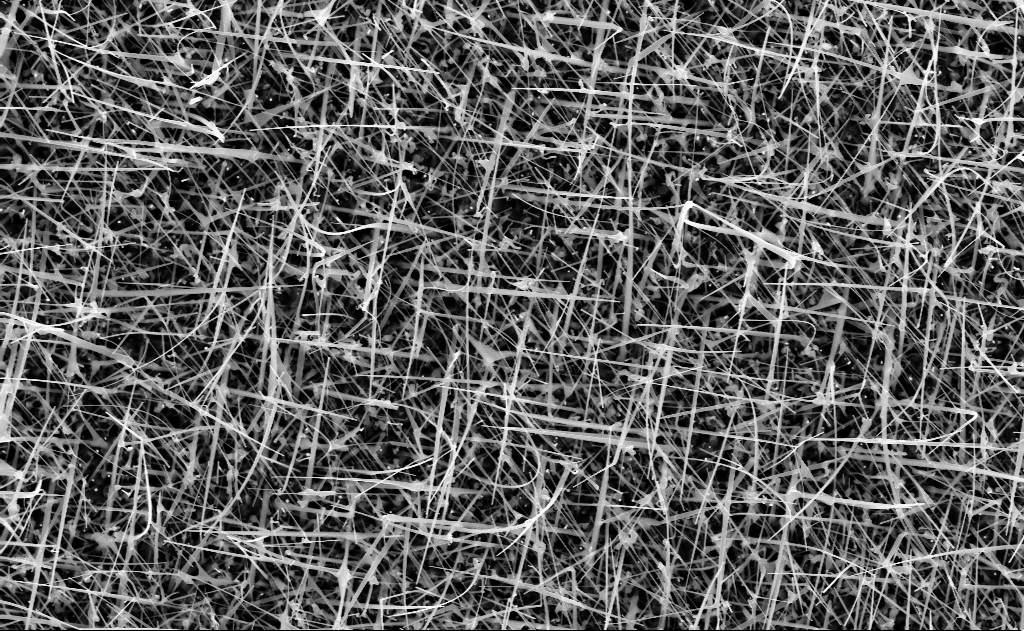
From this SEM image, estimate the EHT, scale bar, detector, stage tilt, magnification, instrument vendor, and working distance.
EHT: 10 kV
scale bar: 2000 nm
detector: InLens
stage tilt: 0°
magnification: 20 K X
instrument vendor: Zeiss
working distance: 9 mm